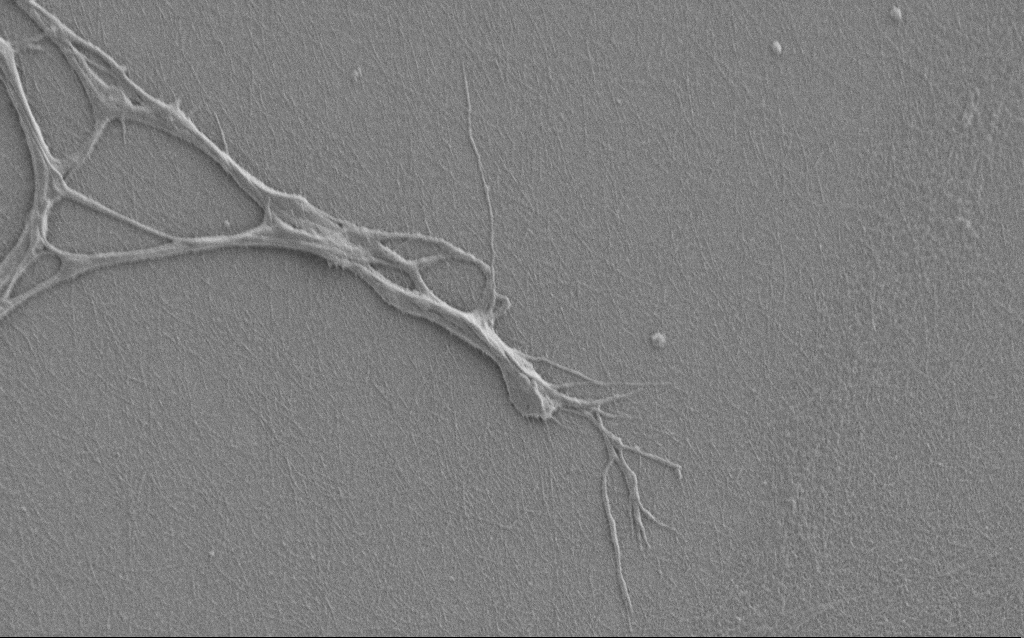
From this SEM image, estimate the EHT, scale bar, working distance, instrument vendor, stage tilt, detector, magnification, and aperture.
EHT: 1 kV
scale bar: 2000 nm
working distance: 6 mm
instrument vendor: Zeiss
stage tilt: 0°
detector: SE2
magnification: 7.5 K X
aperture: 30 µm